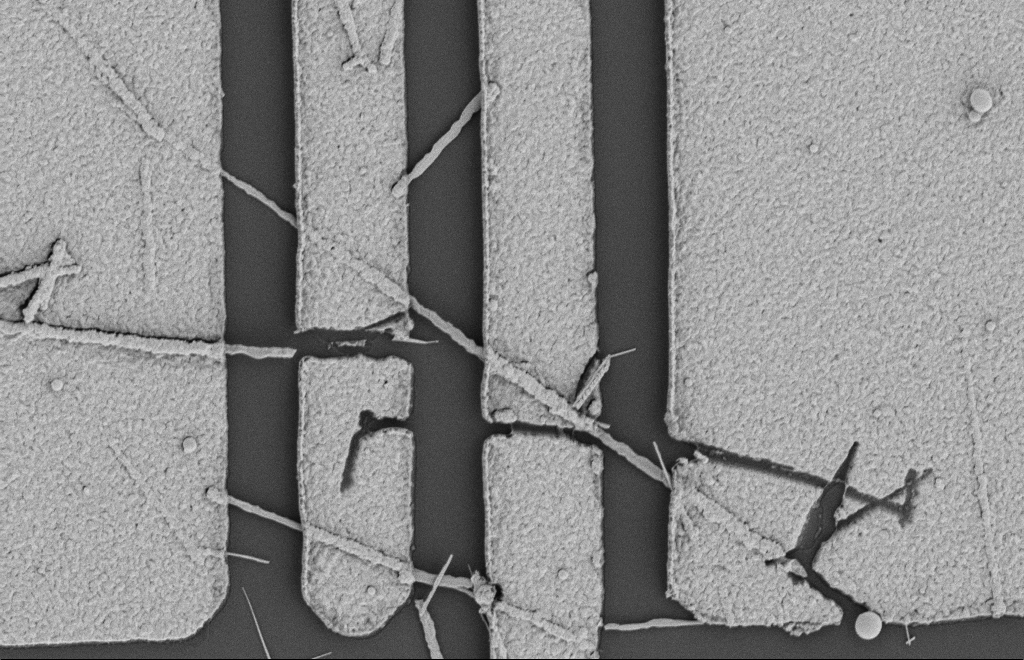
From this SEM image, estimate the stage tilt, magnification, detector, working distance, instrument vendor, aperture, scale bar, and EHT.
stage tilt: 0°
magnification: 16.73 K X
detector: SE2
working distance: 12 mm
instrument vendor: Zeiss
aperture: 20 µm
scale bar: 2000 nm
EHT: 2 kV